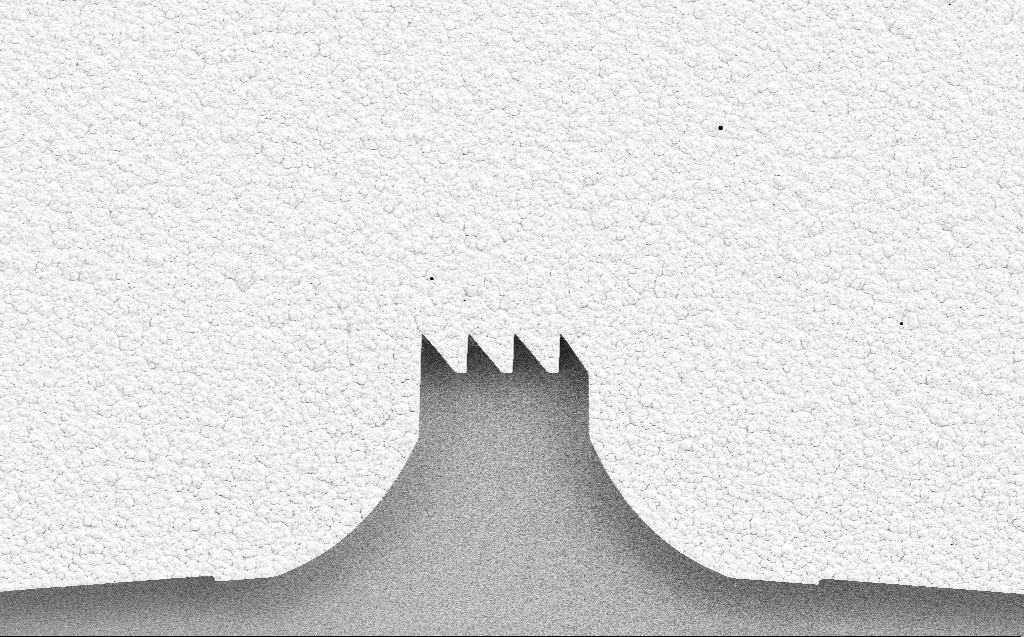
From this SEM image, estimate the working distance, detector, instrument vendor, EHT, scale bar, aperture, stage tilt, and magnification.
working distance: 6 mm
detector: SE2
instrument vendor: Zeiss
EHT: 5 kV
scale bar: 20000 nm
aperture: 30 µm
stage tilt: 0°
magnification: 1.49 K X